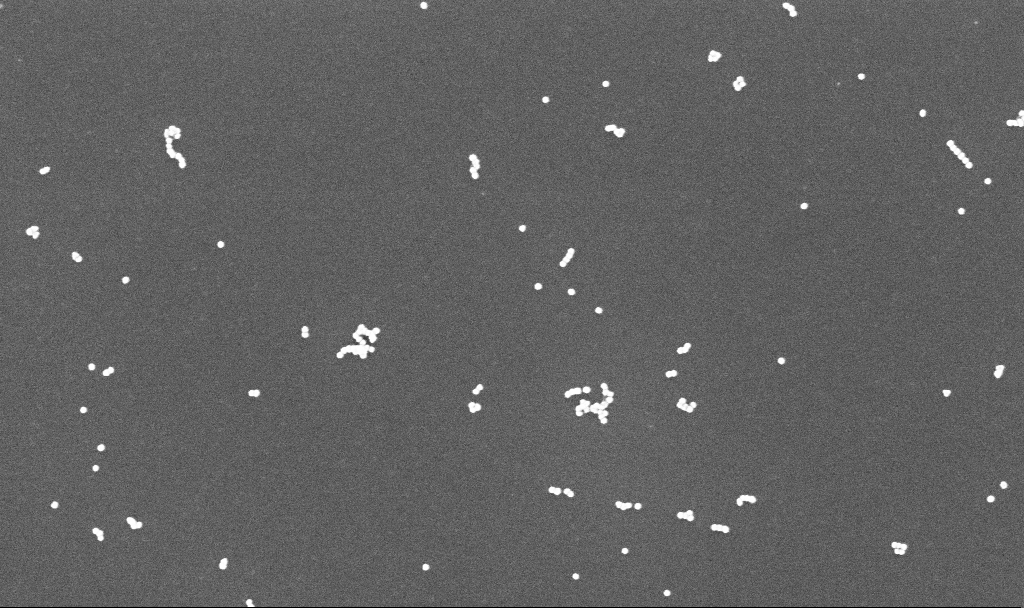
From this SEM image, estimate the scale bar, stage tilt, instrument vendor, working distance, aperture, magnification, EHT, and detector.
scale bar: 200 nm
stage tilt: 0°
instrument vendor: Zeiss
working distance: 3.4 mm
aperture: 30 µm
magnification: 100 K X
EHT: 10 kV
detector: InLens